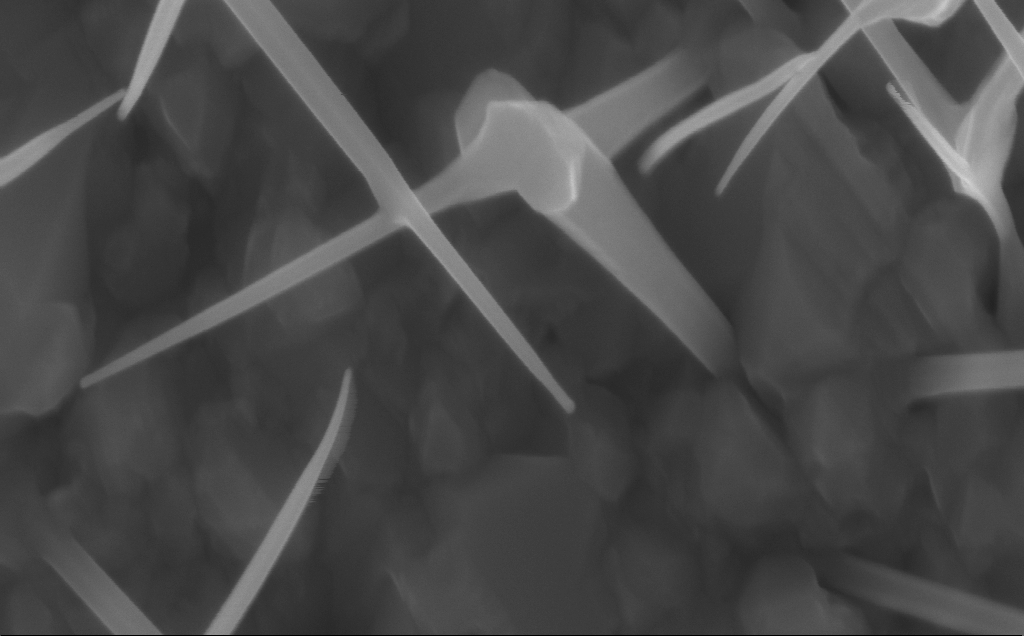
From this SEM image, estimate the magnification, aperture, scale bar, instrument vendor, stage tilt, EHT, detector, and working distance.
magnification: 150 K X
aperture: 30 µm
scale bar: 200 nm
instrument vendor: Zeiss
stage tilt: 0°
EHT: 10 kV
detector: InLens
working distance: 5 mm